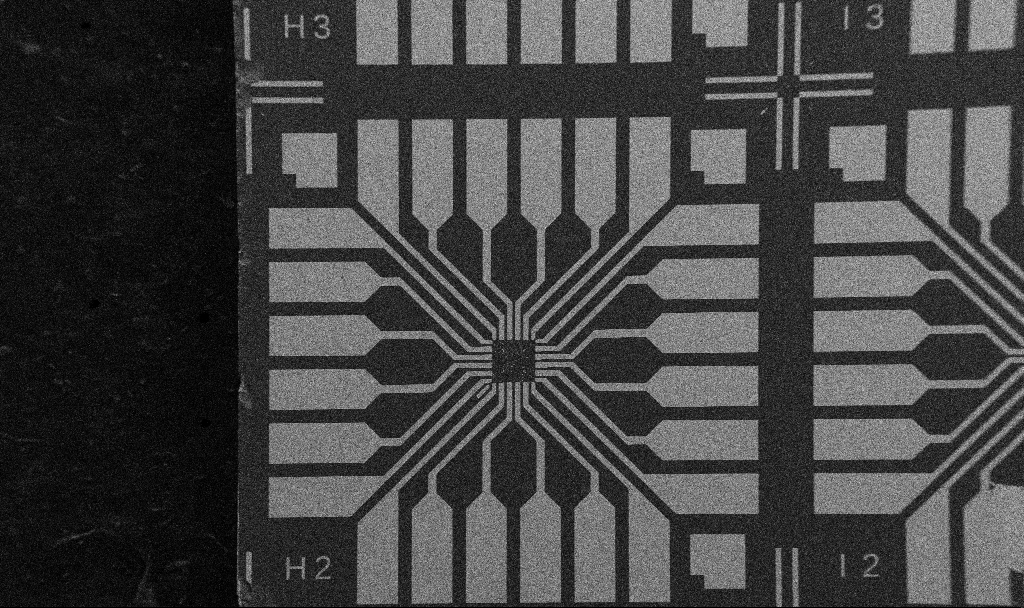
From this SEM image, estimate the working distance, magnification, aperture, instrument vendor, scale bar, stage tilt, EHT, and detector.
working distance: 10.7 mm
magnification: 0.1 K X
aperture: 30 µm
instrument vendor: Zeiss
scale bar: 200000 nm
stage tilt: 0°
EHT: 5 kV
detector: SE2